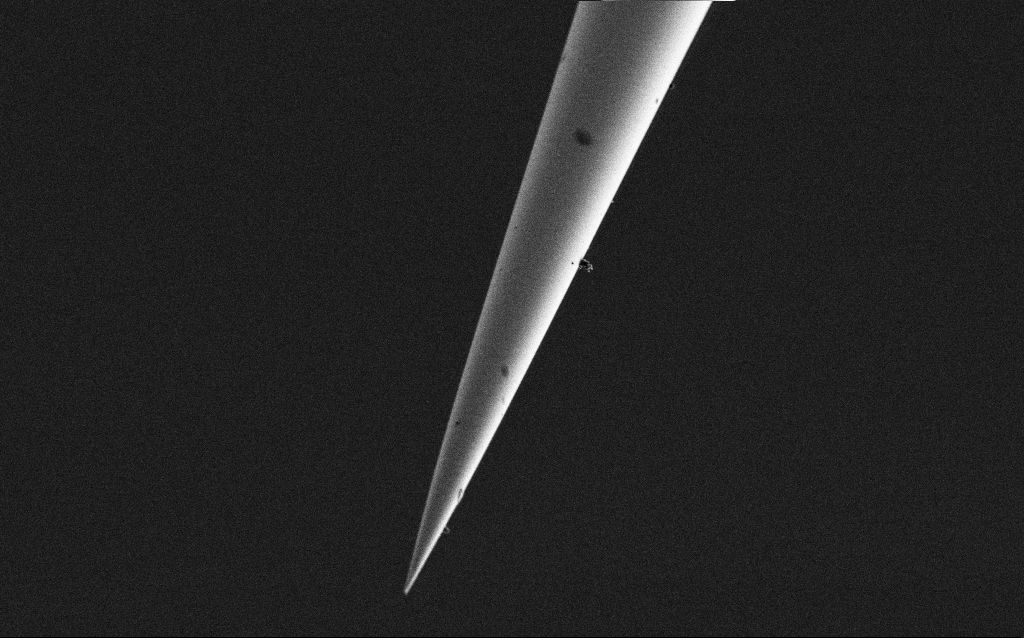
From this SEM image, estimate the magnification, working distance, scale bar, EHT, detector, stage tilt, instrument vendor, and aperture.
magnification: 10 K X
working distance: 7.4 mm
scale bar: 2000 nm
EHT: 1 kV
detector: SE2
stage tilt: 45°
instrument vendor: Zeiss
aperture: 30 µm